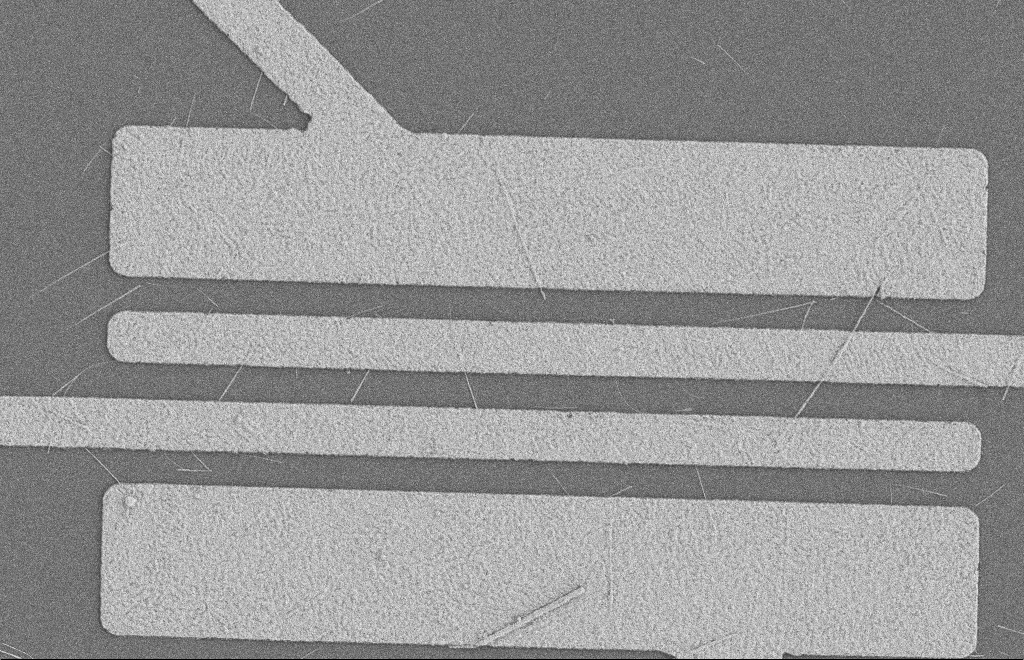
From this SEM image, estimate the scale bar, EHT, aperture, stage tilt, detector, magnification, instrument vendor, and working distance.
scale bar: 2000 nm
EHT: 2 kV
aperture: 20 µm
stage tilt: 0°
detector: SE2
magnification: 5.28 K X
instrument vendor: Zeiss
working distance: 8 mm